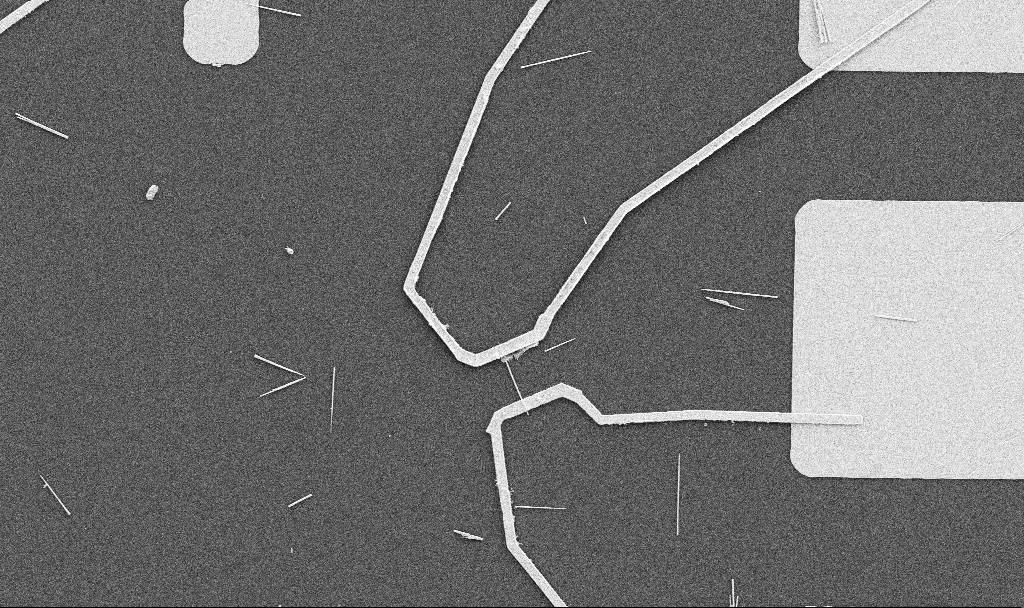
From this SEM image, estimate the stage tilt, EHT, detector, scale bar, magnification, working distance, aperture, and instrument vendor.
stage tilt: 0°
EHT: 5 kV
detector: SE2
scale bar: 10000 nm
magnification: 5 K X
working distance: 10.7 mm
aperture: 30 µm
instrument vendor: Zeiss